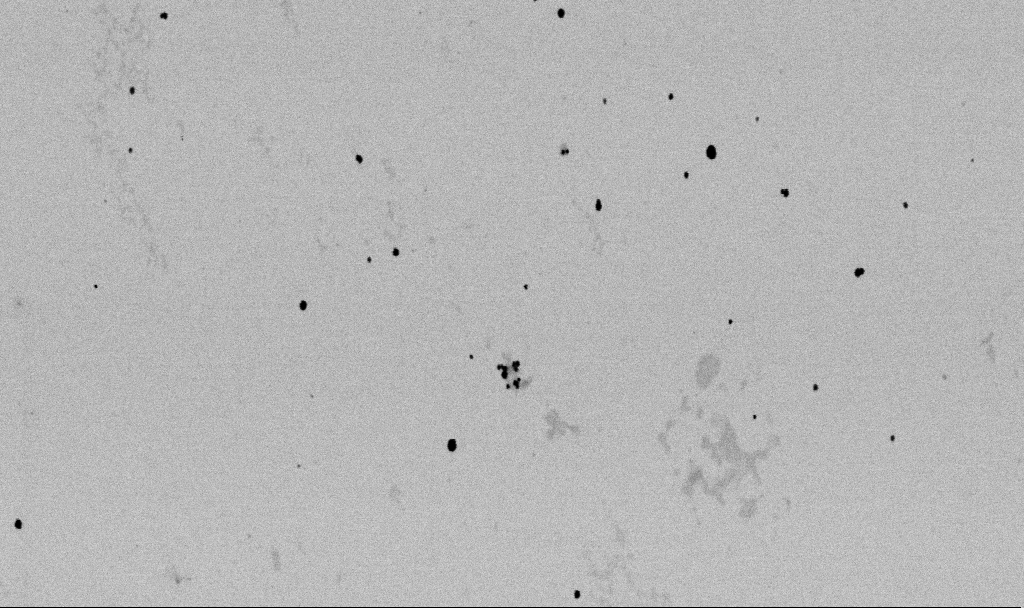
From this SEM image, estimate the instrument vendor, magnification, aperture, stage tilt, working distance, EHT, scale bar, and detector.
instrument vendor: Zeiss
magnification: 50 K X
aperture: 30 µm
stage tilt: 0°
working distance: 6.6 mm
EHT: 2 kV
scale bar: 1000 nm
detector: SE2